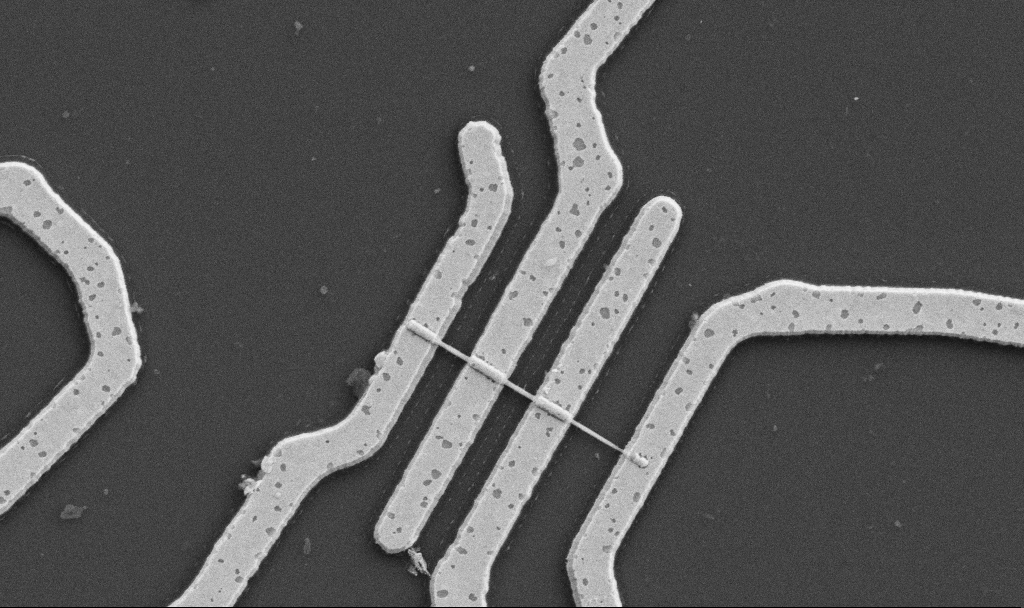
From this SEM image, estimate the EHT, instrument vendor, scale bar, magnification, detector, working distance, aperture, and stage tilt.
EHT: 5 kV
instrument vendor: Zeiss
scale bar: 1000 nm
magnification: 20 K X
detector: SE2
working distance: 10.7 mm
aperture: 30 µm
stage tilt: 0°